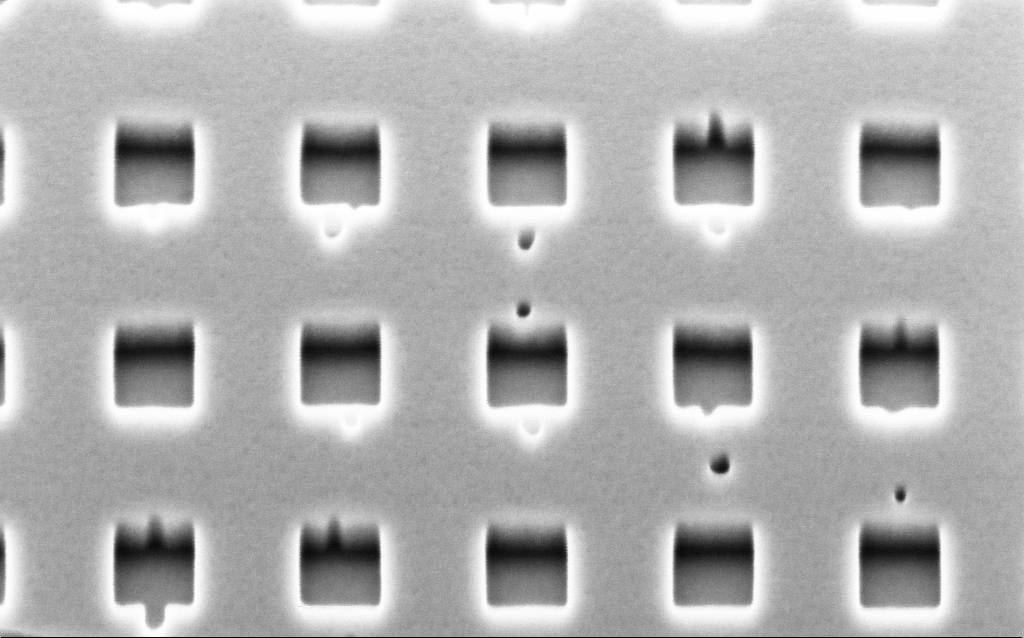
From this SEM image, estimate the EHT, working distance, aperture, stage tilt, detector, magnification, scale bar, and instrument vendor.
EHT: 2 kV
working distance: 3.3 mm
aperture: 30 µm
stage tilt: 45°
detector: InLens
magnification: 137.59 K X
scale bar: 200 nm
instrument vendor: Zeiss